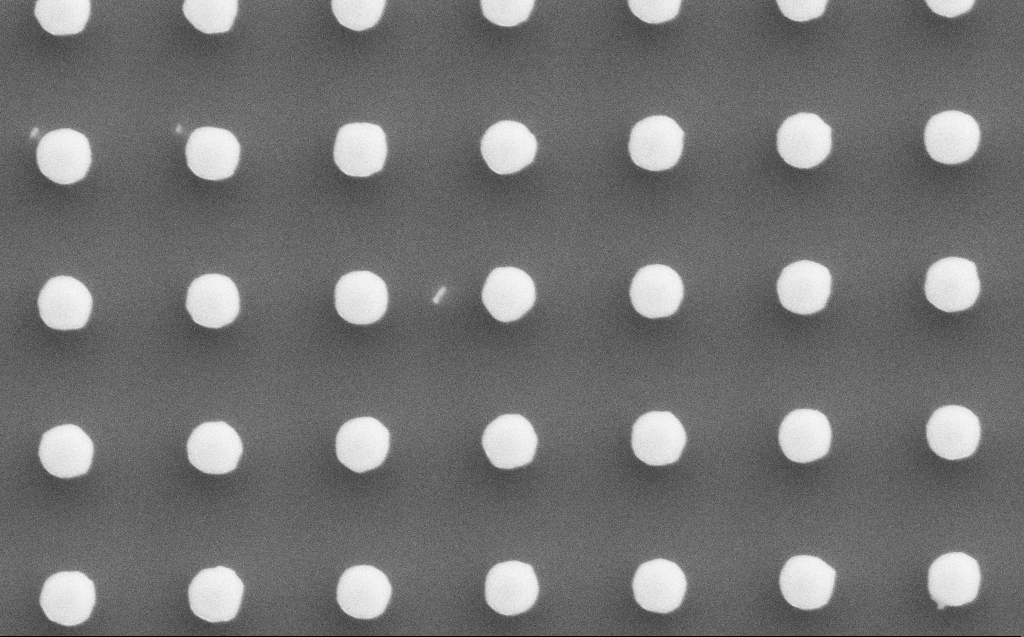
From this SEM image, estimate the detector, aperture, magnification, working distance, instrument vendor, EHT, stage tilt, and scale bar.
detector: SE2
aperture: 30 µm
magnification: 51.87 K X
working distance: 5 mm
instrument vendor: Zeiss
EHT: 5 kV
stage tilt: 0°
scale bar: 1000 nm